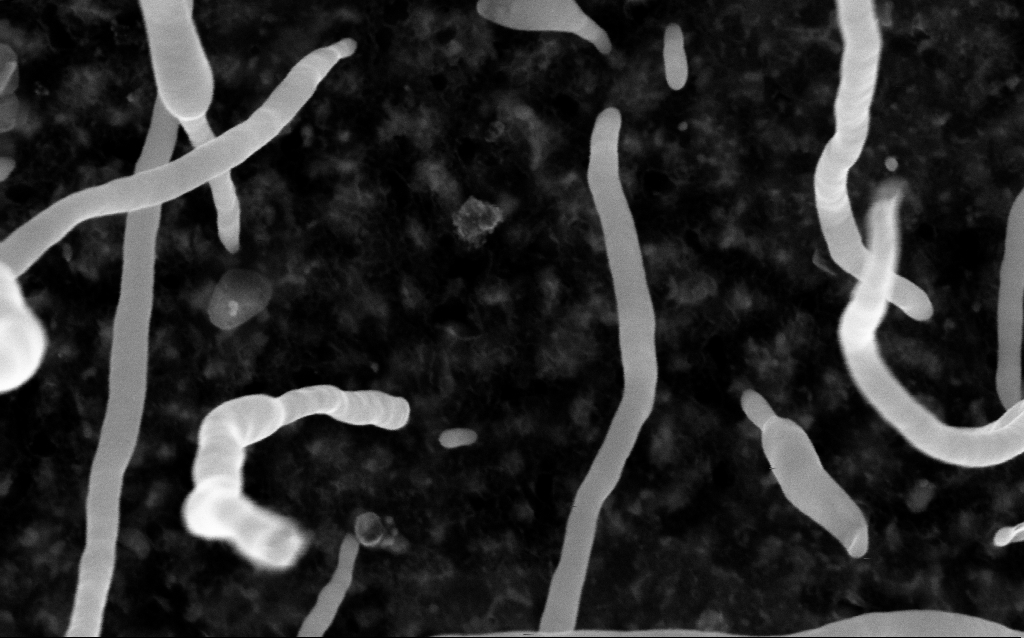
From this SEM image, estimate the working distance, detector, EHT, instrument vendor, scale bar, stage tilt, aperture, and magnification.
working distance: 2.1 mm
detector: InLens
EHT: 5 kV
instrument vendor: Zeiss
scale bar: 100 nm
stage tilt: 0°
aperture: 30 µm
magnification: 200 K X